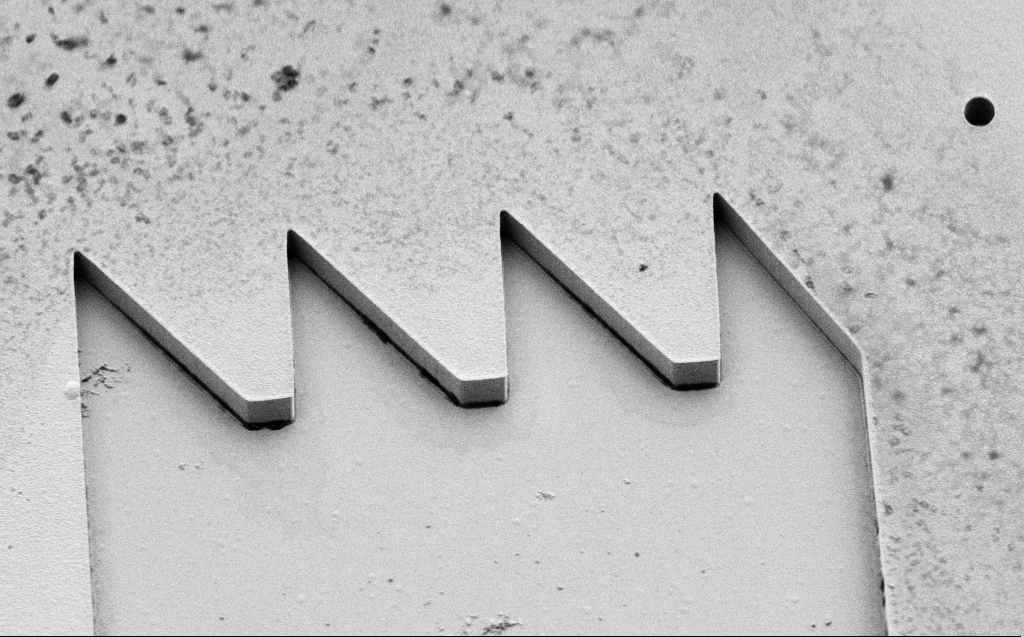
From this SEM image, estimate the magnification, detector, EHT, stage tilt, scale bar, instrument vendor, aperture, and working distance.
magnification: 6.86 K X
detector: SE2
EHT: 3 kV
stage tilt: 45.1°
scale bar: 10000 nm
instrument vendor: Zeiss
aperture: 30 µm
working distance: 9 mm